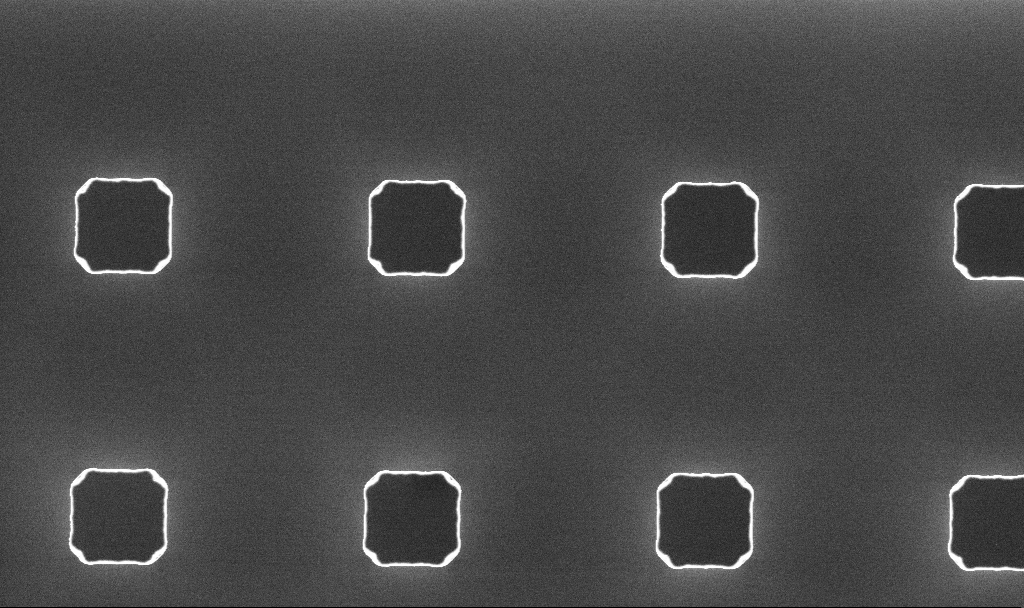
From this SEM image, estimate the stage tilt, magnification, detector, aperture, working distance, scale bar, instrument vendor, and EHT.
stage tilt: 0°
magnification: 3.6 K X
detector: InLens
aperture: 30 µm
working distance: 5.3 mm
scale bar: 10000 nm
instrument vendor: Zeiss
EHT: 5 kV